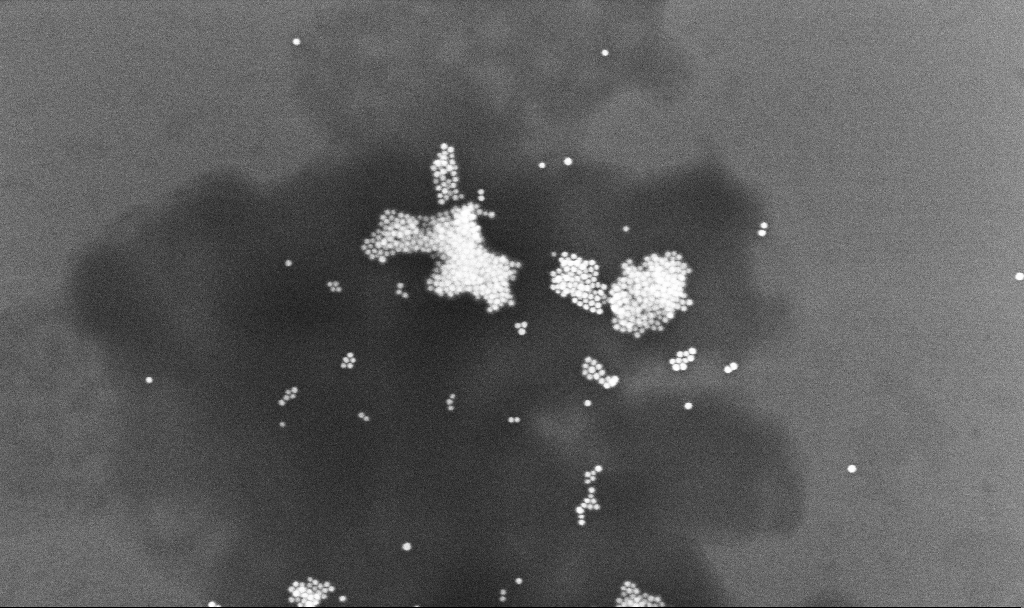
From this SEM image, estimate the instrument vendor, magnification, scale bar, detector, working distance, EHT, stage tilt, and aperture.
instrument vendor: Zeiss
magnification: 108.67 K X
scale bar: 200 nm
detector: InLens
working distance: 3.3 mm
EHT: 10 kV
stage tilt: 0°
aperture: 30 µm